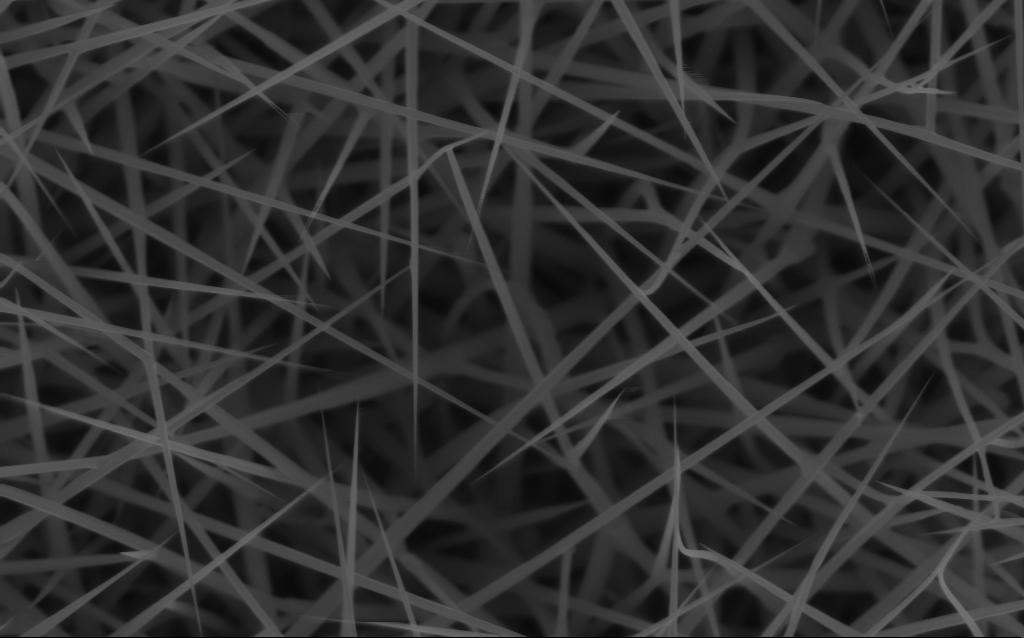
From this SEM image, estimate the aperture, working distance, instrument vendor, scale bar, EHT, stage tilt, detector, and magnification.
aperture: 30 µm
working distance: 6 mm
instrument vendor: Zeiss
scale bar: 1000 nm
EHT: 10 kV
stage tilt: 0°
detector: InLens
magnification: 40 K X